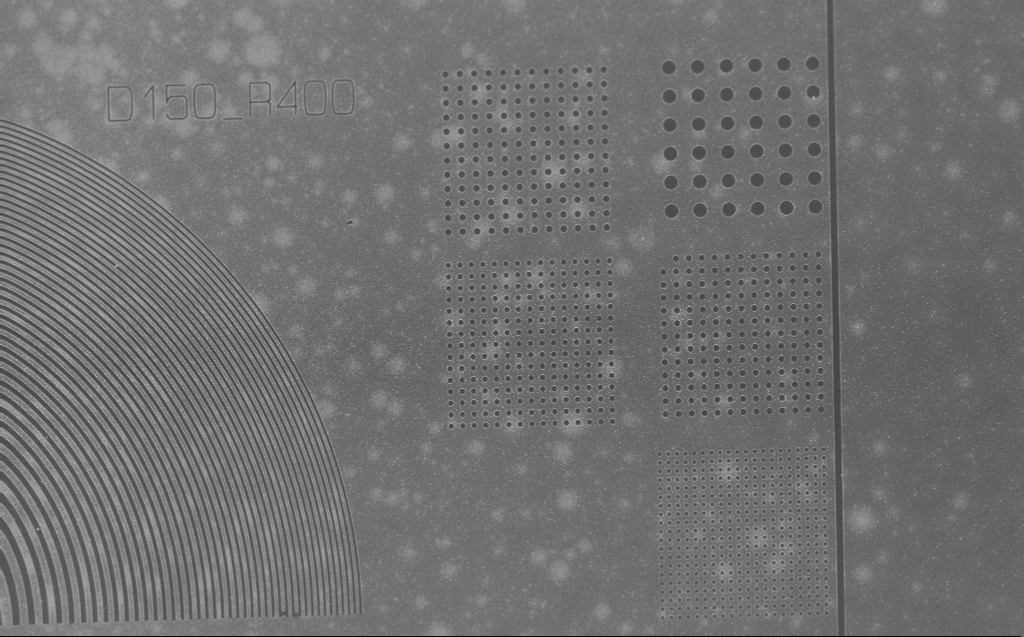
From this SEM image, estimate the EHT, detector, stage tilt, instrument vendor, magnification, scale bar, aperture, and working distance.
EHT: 2.5 kV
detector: InLens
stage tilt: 30°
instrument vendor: Zeiss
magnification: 2.68 K X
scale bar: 10000 nm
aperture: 30 µm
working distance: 4 mm